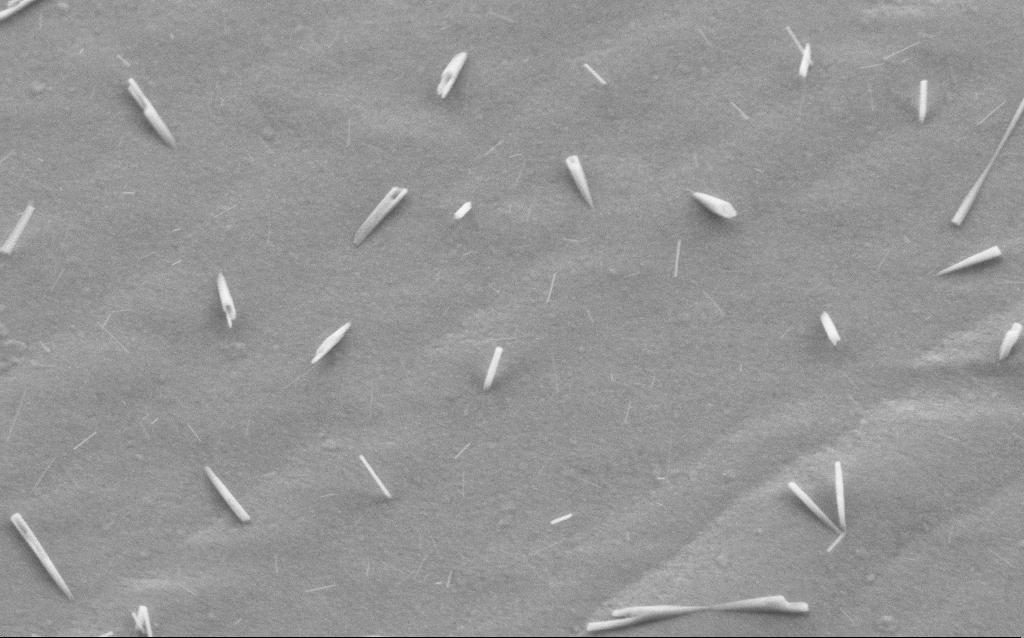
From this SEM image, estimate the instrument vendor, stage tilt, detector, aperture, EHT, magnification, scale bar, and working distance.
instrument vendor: Zeiss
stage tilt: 23.6°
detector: SE2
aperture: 30 µm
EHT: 5 kV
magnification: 46.99 K X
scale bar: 1000 nm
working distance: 3 mm